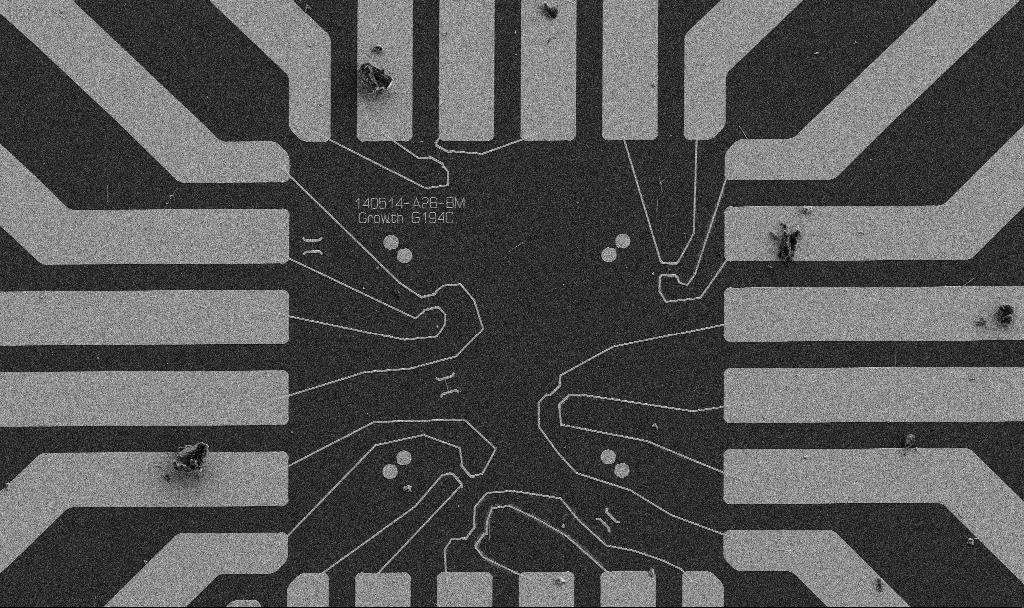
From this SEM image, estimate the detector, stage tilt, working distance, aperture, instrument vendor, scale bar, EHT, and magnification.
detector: SE2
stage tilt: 0°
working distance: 10.7 mm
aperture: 30 µm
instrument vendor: Zeiss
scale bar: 20000 nm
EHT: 5 kV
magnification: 1 K X